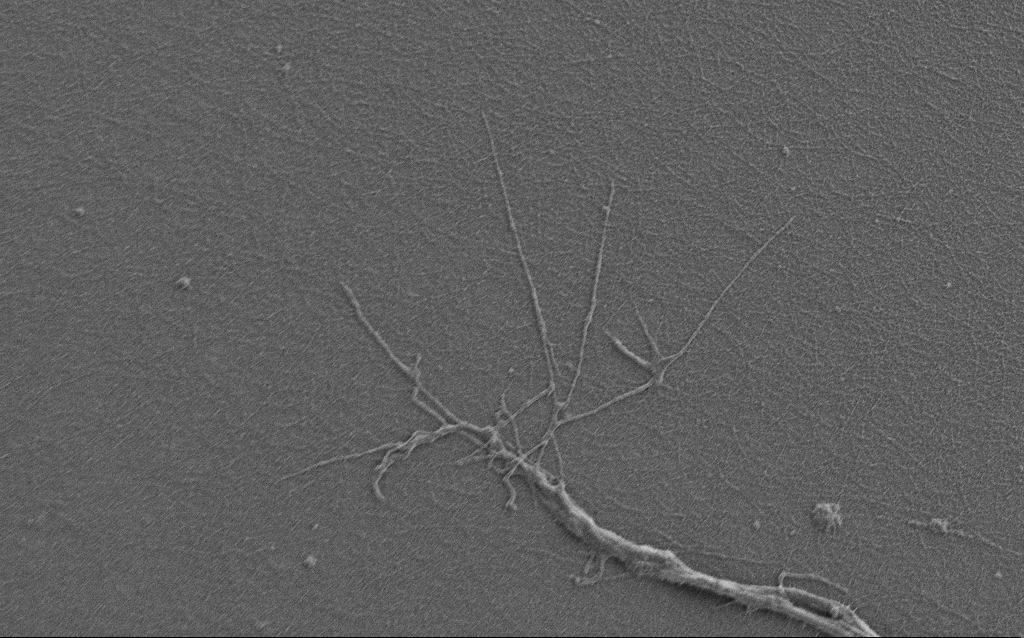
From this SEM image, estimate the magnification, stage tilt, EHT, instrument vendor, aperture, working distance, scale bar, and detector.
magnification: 7.5 K X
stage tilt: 0°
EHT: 0.9 kV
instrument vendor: Zeiss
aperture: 30 µm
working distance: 6 mm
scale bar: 2000 nm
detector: SE2